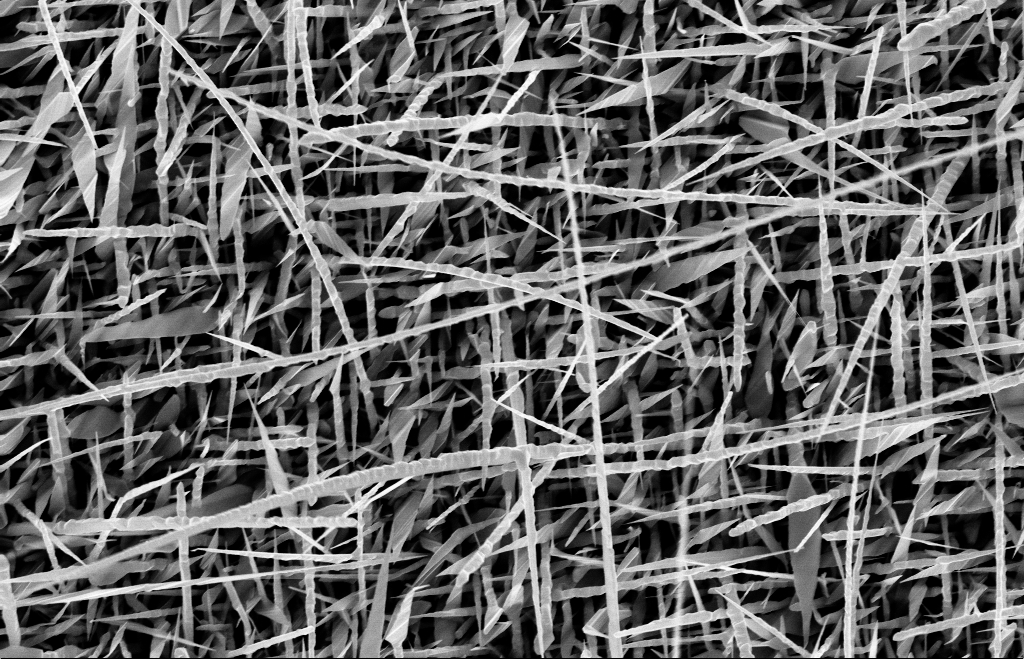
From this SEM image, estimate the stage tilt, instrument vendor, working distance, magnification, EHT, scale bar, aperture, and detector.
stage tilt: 0°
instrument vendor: Zeiss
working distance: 9 mm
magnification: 20 K X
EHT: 10 kV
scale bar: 1000 nm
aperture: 30 µm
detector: InLens